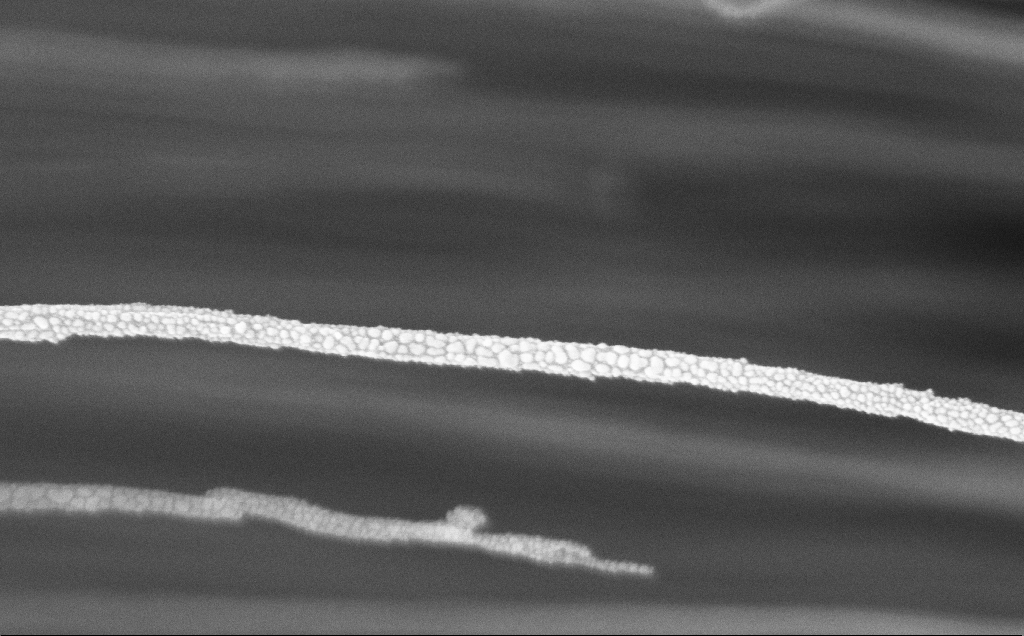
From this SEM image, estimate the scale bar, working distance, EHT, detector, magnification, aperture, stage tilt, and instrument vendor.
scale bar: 200 nm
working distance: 12 mm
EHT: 5 kV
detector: InLens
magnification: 89.56 K X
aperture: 30 µm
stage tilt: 0°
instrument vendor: Zeiss